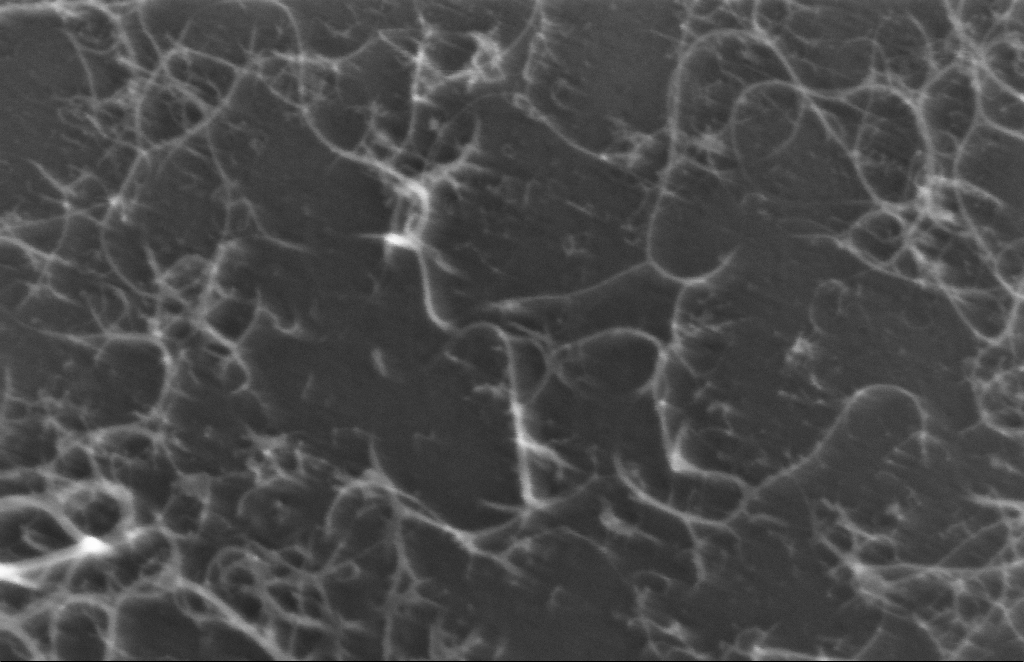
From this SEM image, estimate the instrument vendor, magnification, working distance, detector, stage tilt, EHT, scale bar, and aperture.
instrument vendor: Zeiss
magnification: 416.5 K X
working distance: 5 mm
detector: InLens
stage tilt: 0°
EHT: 5 kV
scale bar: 100 nm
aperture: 30 µm